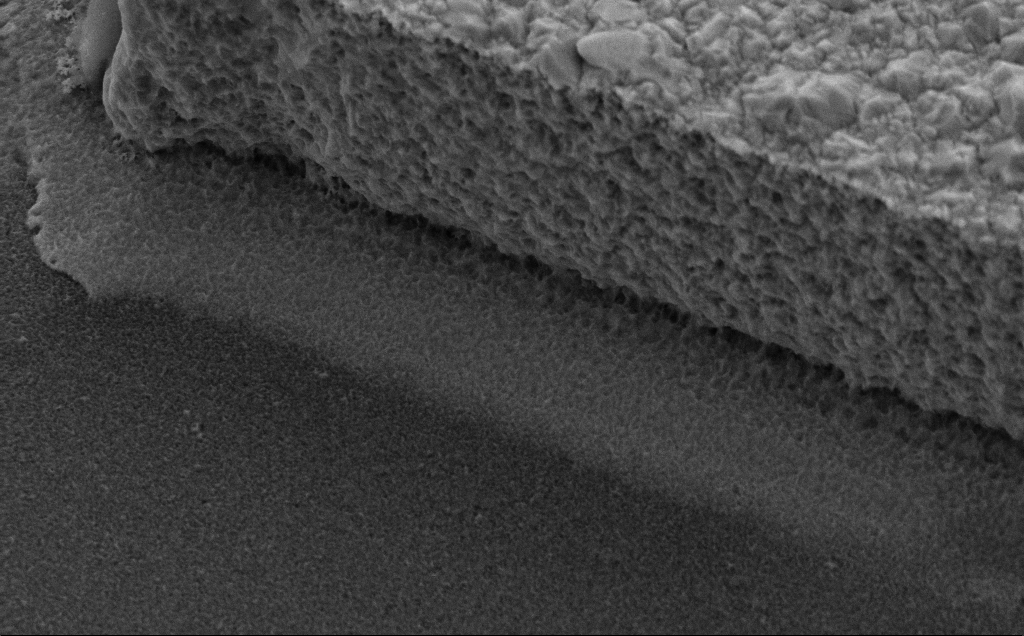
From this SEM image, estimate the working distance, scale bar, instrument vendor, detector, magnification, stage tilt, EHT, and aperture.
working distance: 8 mm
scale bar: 2000 nm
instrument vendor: Zeiss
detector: SE2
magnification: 30.42 K X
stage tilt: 35°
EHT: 10 kV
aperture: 30 µm